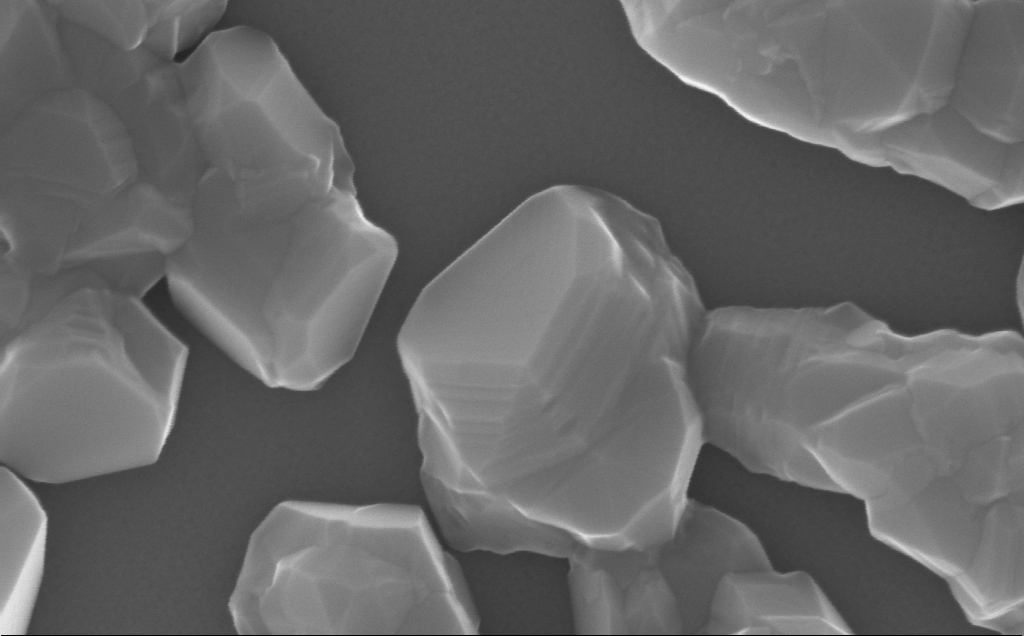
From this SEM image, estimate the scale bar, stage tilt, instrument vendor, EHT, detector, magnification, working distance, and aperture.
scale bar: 200 nm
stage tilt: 0°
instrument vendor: Zeiss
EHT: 10 kV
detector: InLens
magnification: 208.94 K X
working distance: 6 mm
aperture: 30 µm